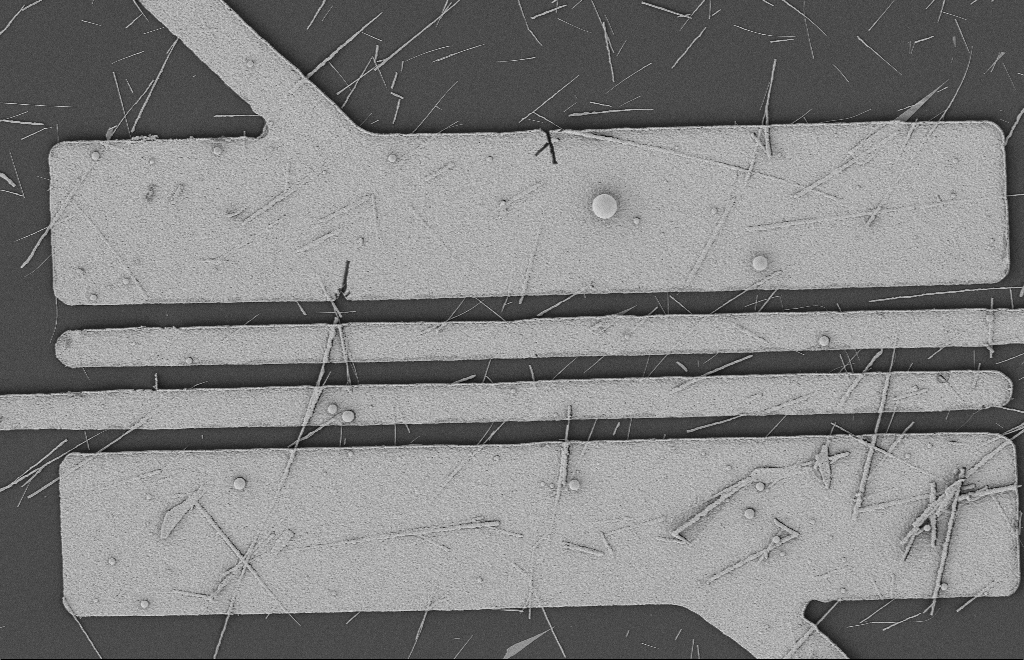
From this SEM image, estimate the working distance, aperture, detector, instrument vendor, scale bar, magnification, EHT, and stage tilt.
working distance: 12 mm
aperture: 20 µm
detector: SE2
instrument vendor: Zeiss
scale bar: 2000 nm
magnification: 5.71 K X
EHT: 2 kV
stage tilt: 0°